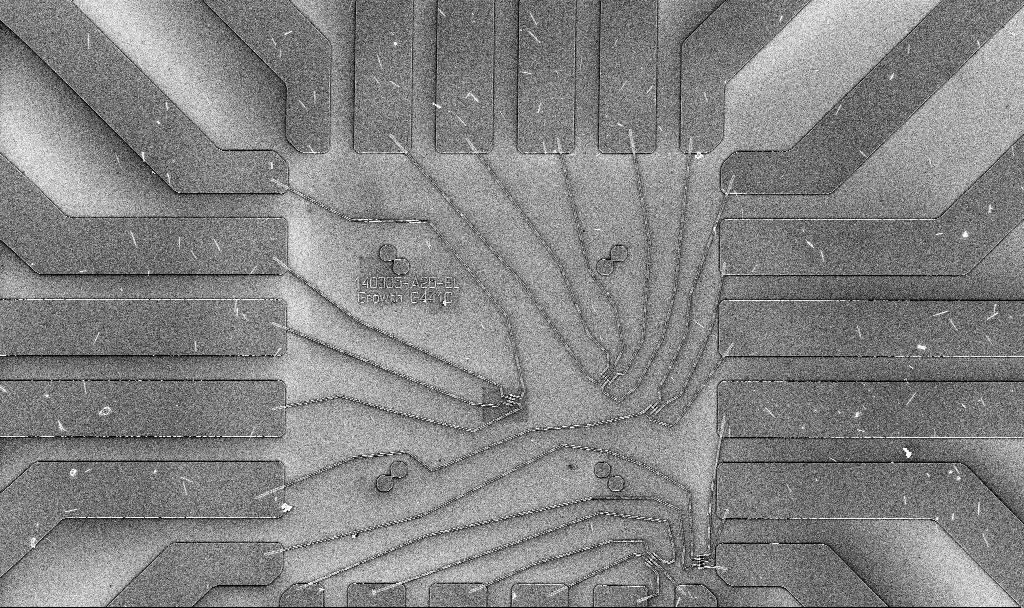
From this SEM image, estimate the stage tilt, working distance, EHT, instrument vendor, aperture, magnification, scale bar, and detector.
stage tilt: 0°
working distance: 6.7 mm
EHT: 10 kV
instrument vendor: Zeiss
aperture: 30 µm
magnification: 1 K X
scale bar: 20000 nm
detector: InLens